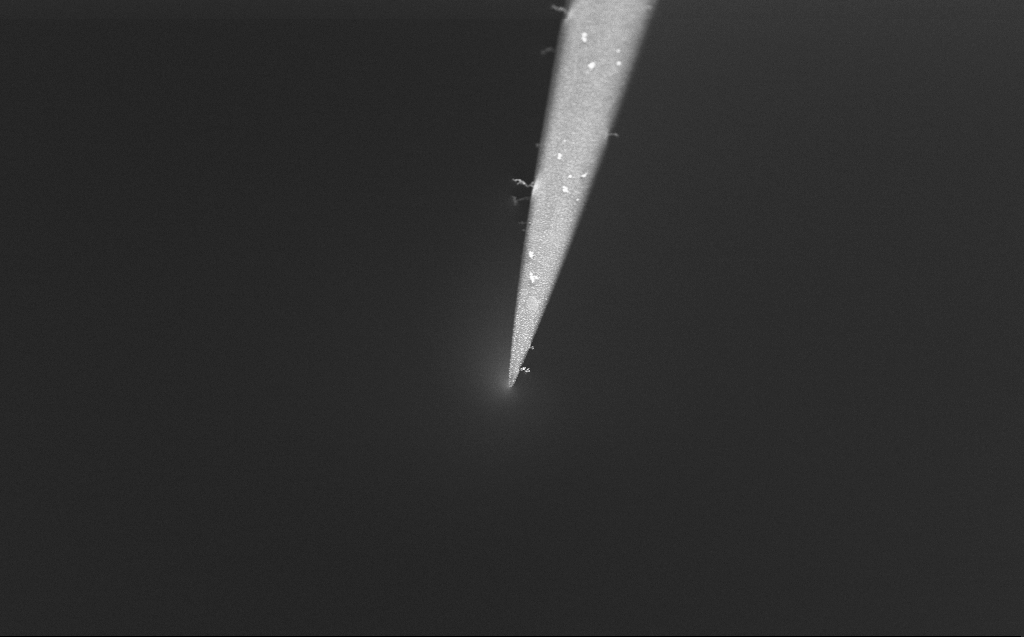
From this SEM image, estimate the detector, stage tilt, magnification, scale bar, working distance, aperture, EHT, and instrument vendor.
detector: InLens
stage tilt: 45°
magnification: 10 K X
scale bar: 2000 nm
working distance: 3 mm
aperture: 30 µm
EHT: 5 kV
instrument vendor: Zeiss